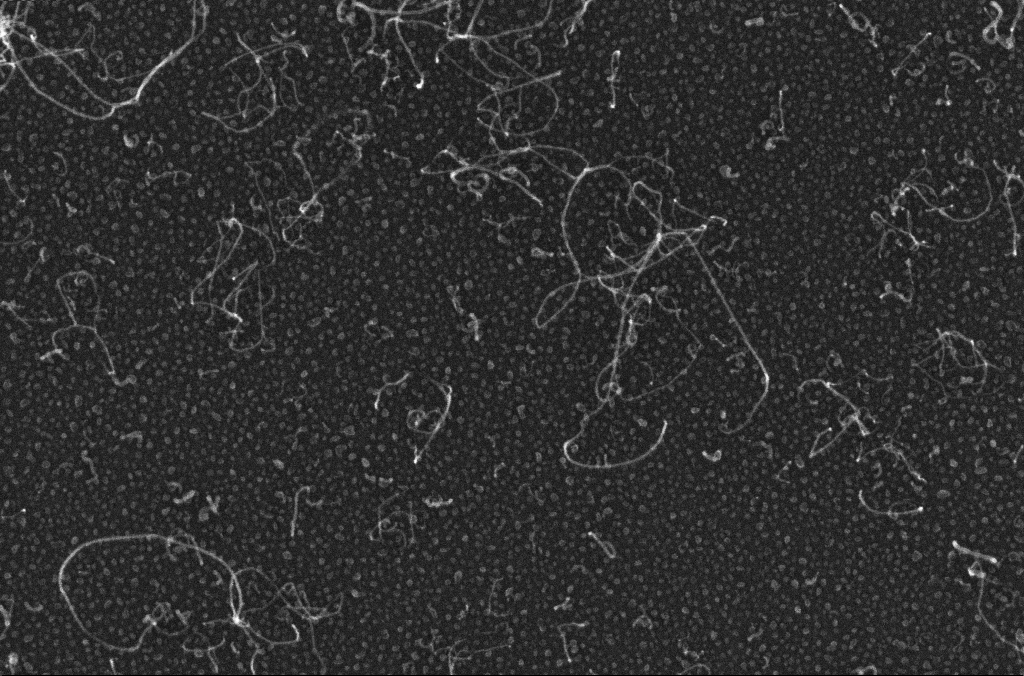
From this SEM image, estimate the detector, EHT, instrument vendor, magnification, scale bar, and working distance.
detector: InLens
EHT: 10 kV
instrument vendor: Zeiss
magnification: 150 K X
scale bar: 100 nm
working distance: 3.2 mm